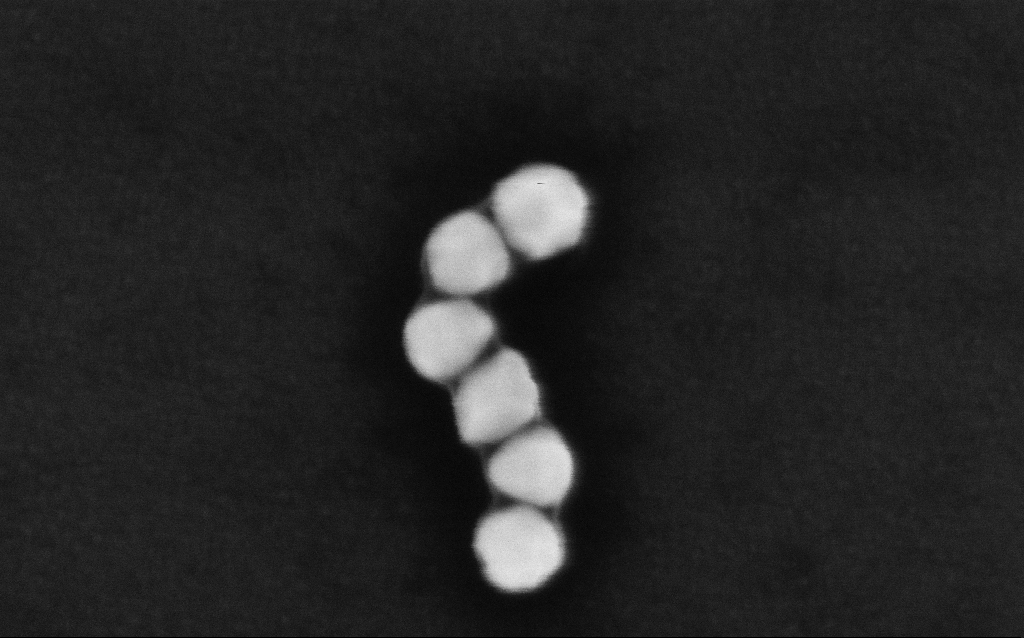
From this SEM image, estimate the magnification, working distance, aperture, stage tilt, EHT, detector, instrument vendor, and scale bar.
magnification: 499.22 K X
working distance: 3.2 mm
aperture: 30 µm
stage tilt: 0°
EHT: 8 kV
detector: InLens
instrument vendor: Zeiss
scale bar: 100 nm